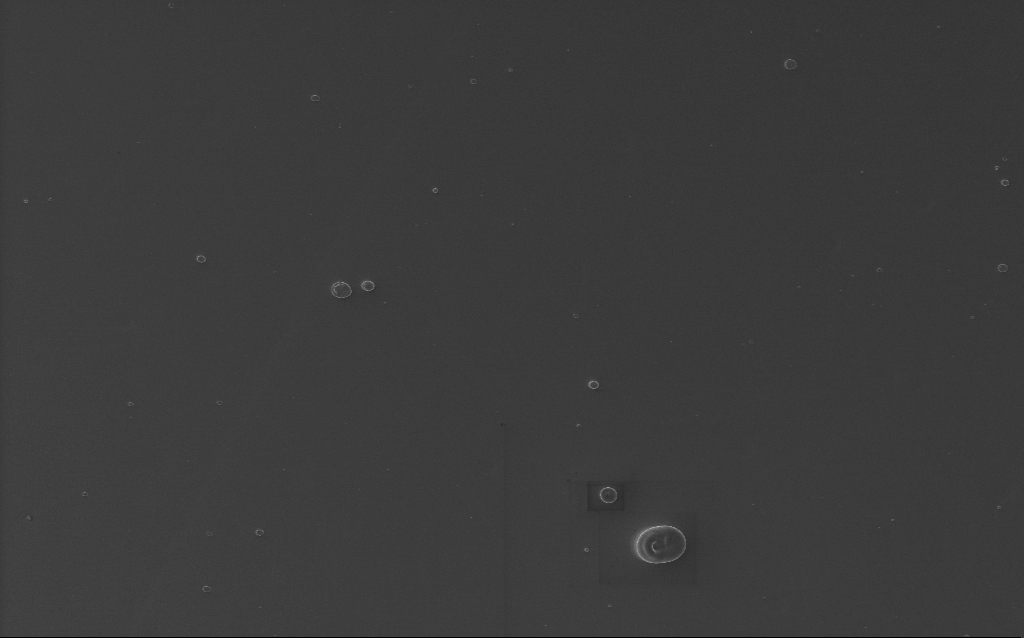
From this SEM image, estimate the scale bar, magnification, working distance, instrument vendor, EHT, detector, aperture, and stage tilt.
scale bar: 10000 nm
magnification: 3.61 K X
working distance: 2 mm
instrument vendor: Zeiss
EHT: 5 kV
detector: InLens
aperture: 30 µm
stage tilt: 0°